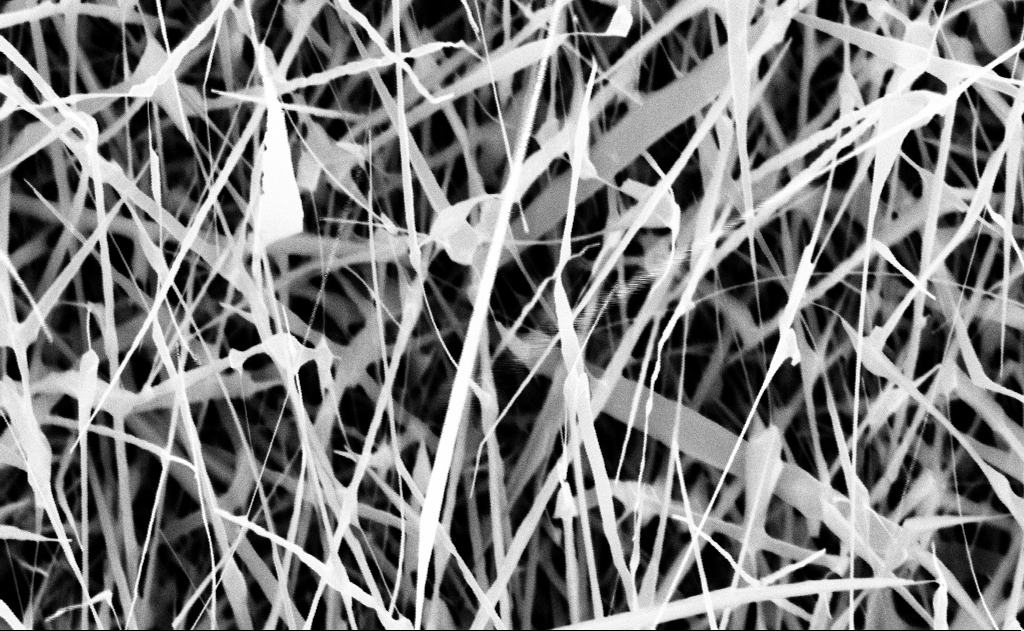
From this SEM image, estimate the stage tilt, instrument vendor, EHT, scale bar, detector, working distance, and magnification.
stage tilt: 0°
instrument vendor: Zeiss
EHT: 10 kV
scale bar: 1000 nm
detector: InLens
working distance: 16 mm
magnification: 40 K X